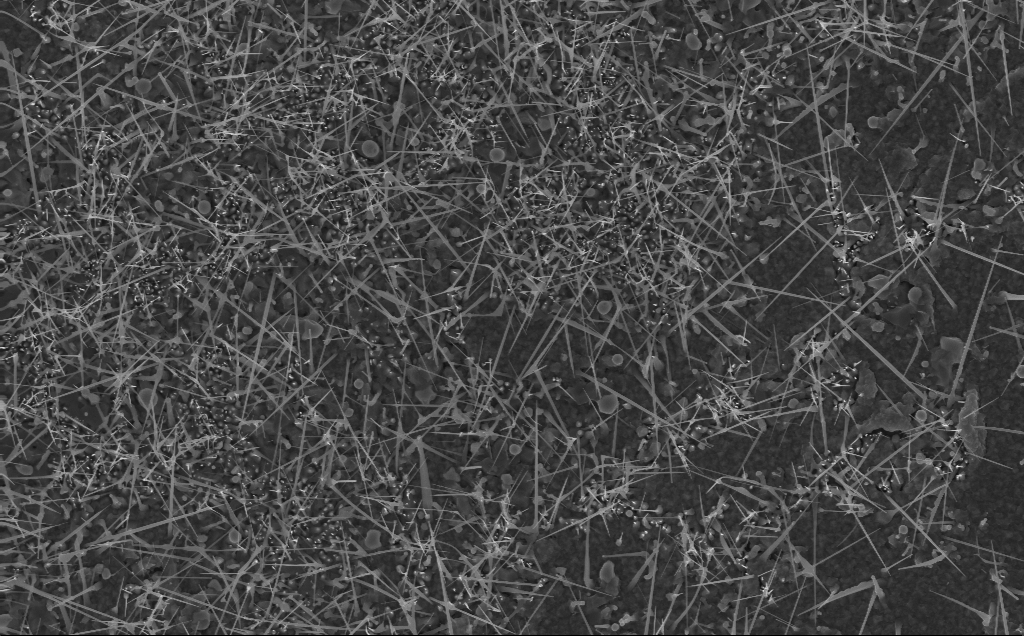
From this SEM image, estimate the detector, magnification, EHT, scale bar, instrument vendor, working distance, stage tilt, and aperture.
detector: InLens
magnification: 8.75 K X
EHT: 10 kV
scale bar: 2000 nm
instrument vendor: Zeiss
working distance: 3 mm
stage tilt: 0°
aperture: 30 µm